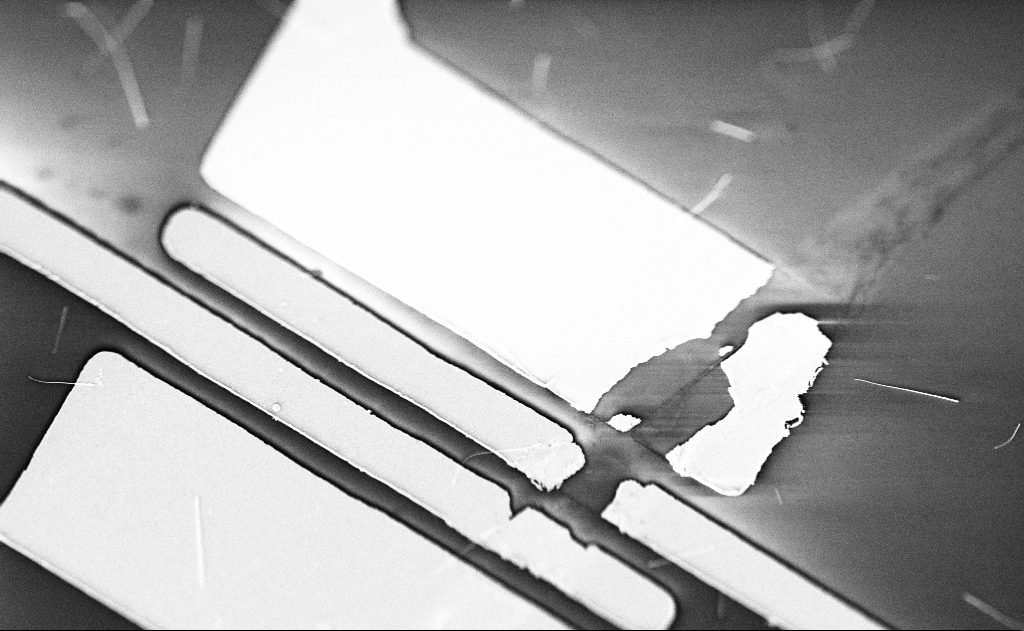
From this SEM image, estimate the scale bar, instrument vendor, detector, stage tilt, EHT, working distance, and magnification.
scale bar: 2000 nm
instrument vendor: Zeiss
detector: SE2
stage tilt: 0°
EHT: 5 kV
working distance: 20 mm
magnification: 7.47 K X